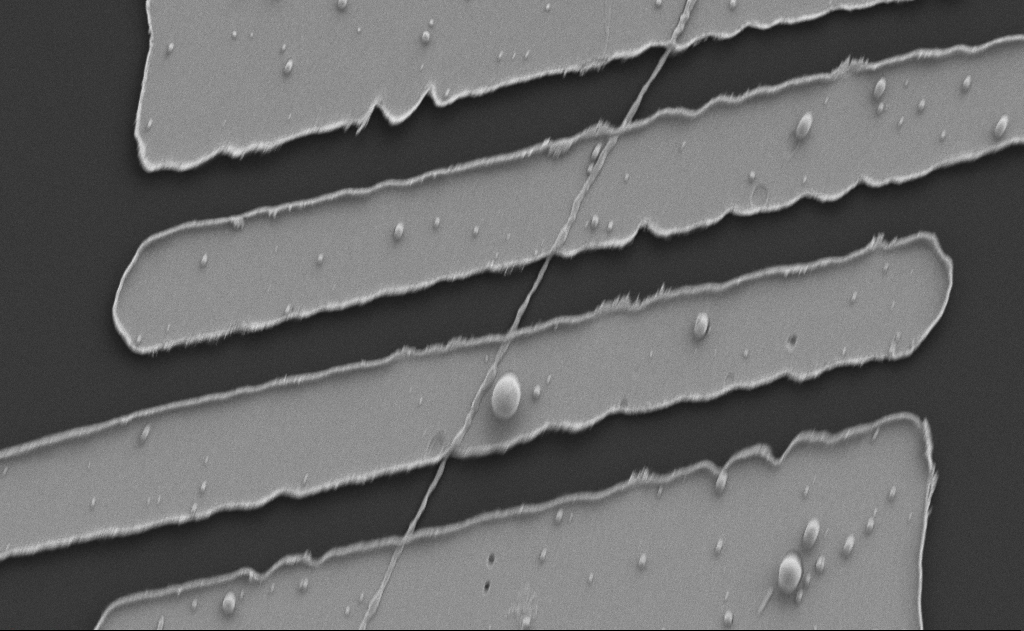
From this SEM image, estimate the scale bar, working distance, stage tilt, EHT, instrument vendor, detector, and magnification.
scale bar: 2000 nm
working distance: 10 mm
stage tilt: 0°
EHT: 5 kV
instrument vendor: Zeiss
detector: SE2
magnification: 10.23 K X